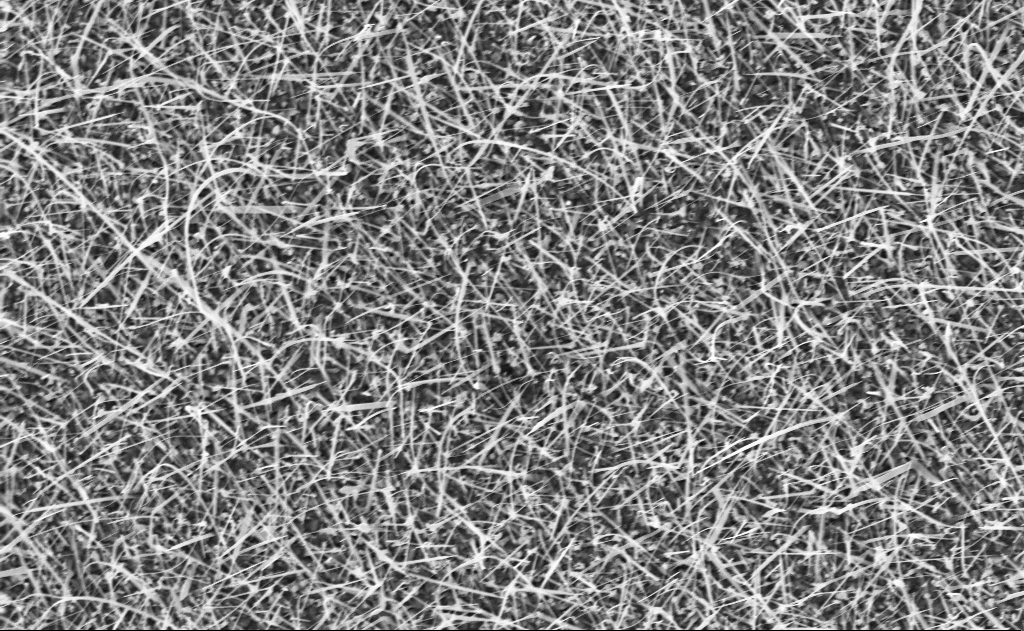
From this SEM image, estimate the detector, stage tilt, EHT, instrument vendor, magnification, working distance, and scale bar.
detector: InLens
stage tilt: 0°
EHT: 10 kV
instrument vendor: Zeiss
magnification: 10 K X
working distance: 11 mm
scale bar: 2000 nm